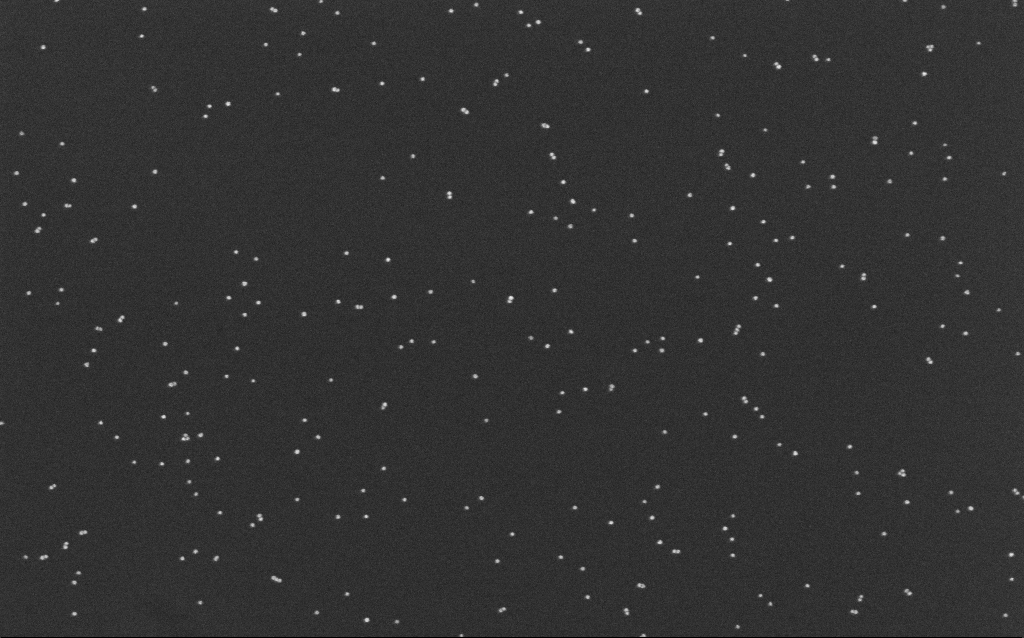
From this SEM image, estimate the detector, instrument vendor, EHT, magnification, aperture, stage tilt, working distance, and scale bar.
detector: InLens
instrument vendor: Zeiss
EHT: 10 kV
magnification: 100 K X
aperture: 30 µm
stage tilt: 0°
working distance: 6.6 mm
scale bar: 200 nm